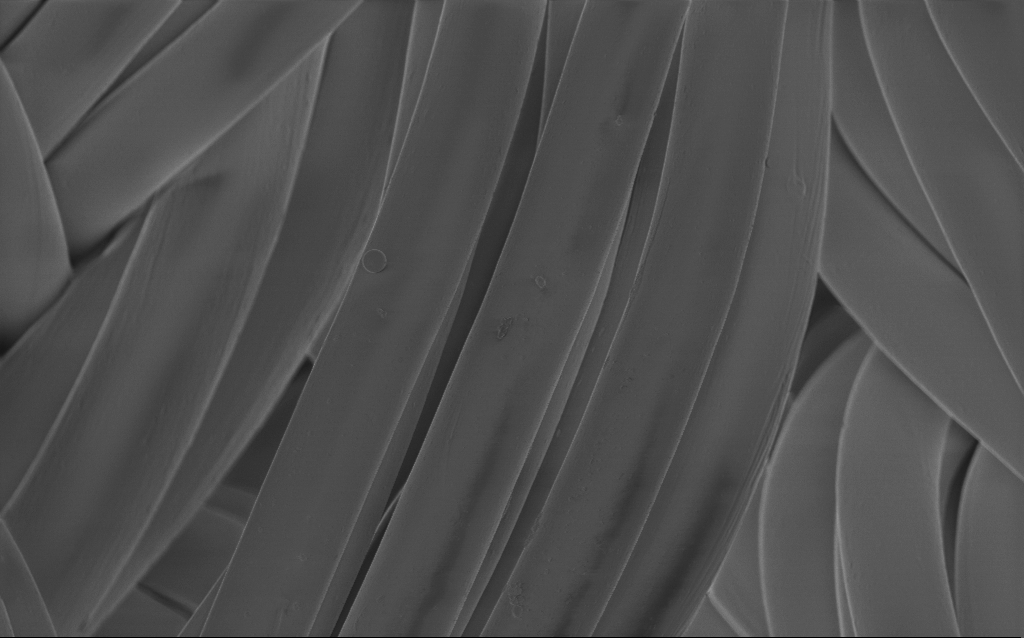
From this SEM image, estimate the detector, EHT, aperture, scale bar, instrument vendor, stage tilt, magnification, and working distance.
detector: InLens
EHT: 1 kV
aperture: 30 µm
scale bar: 20000 nm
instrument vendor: Zeiss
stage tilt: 0°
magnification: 1.58 K X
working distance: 4 mm